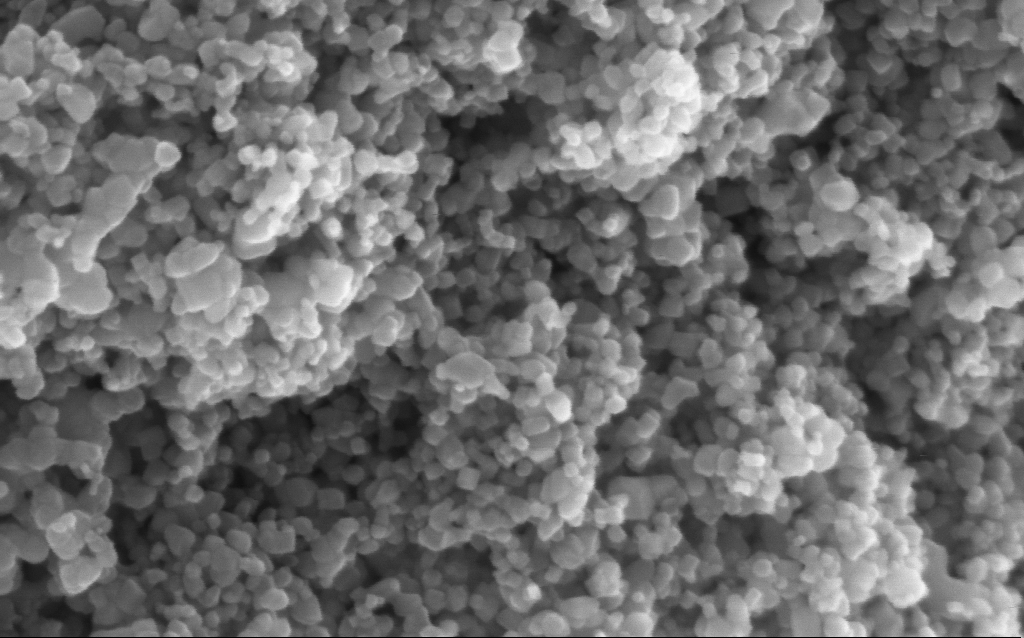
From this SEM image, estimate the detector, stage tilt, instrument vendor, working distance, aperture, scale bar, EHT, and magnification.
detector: InLens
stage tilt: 0°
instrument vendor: Zeiss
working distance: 4 mm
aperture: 30 µm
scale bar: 100 nm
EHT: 5 kV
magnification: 294.04 K X